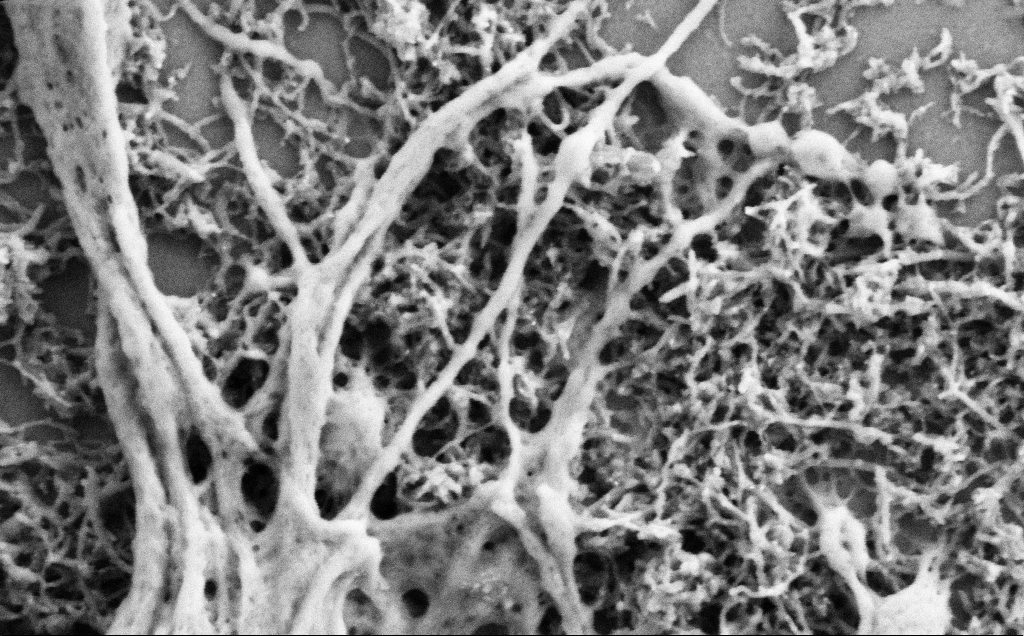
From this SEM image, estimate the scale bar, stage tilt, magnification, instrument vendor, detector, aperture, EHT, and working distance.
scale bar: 200 nm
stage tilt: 0°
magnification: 75 K X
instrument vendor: Zeiss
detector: SE2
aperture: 30 µm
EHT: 2 kV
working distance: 7 mm